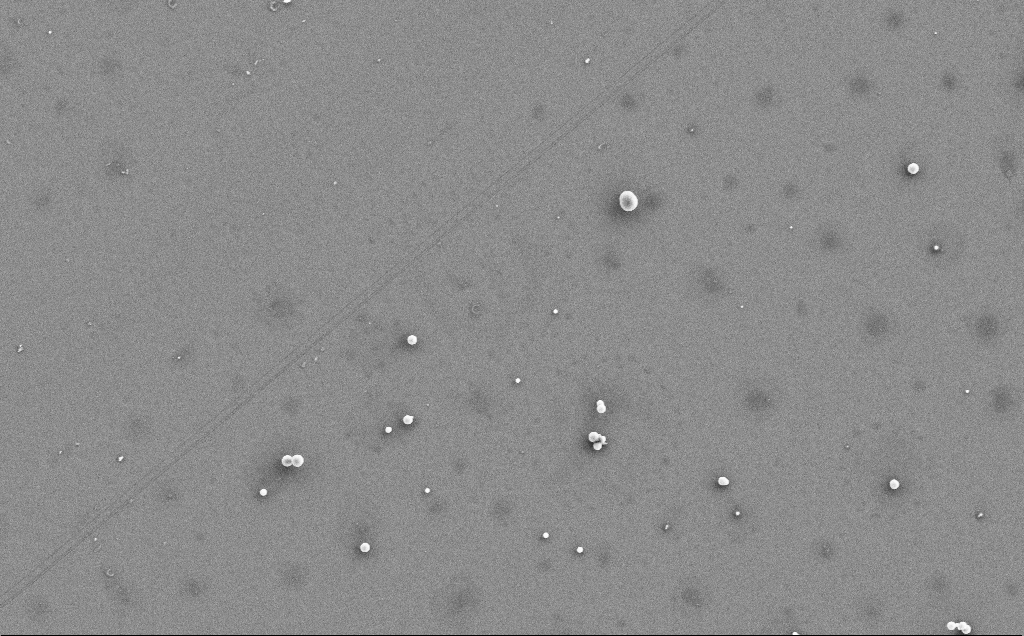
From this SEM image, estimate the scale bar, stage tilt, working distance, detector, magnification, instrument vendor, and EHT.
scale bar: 10000 nm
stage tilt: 0°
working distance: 5 mm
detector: SE2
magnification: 4.01 K X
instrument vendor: Zeiss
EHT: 5 kV